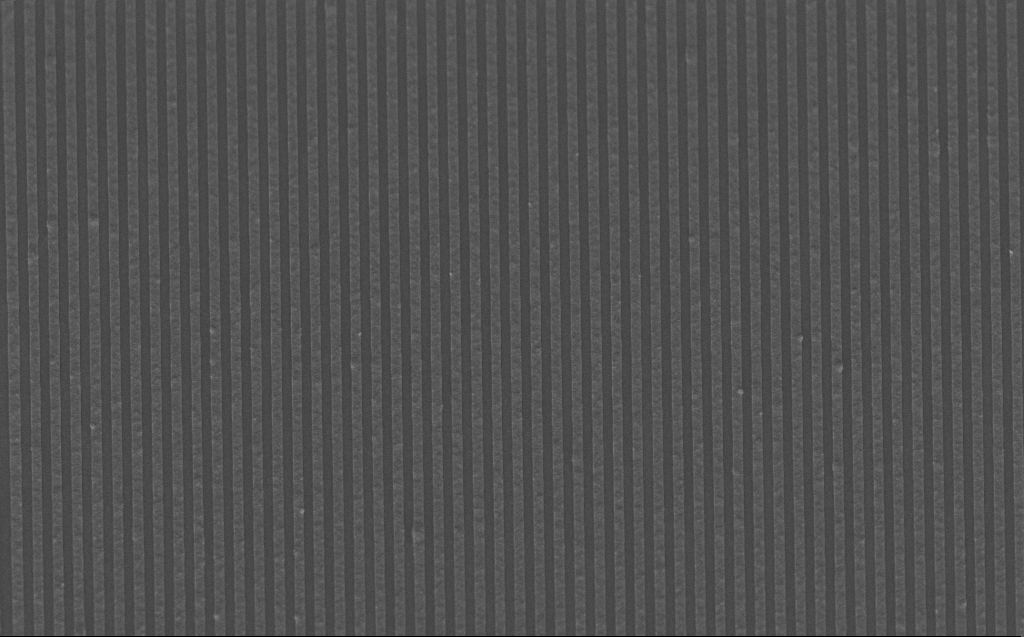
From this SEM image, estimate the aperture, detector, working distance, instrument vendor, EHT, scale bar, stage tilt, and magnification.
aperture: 30 µm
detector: InLens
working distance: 8 mm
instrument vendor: Zeiss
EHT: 5 kV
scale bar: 1000 nm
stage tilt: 45°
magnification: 14.85 K X